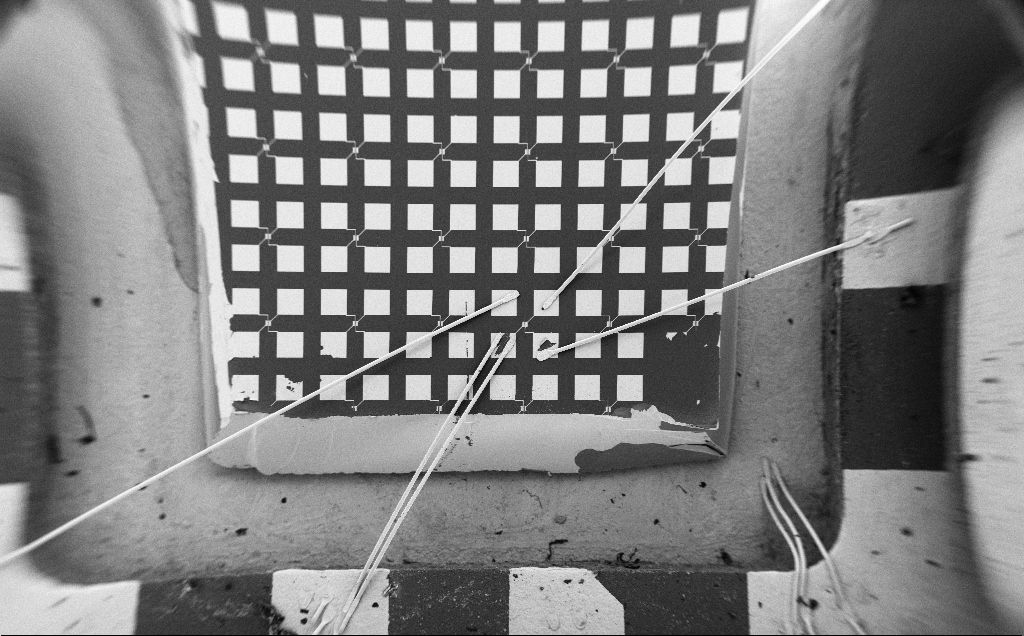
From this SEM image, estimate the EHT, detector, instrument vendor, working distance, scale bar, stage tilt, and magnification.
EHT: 5 kV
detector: SE2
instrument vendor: Zeiss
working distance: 10 mm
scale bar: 1e+06 nm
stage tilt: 0°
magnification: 0.064 K X